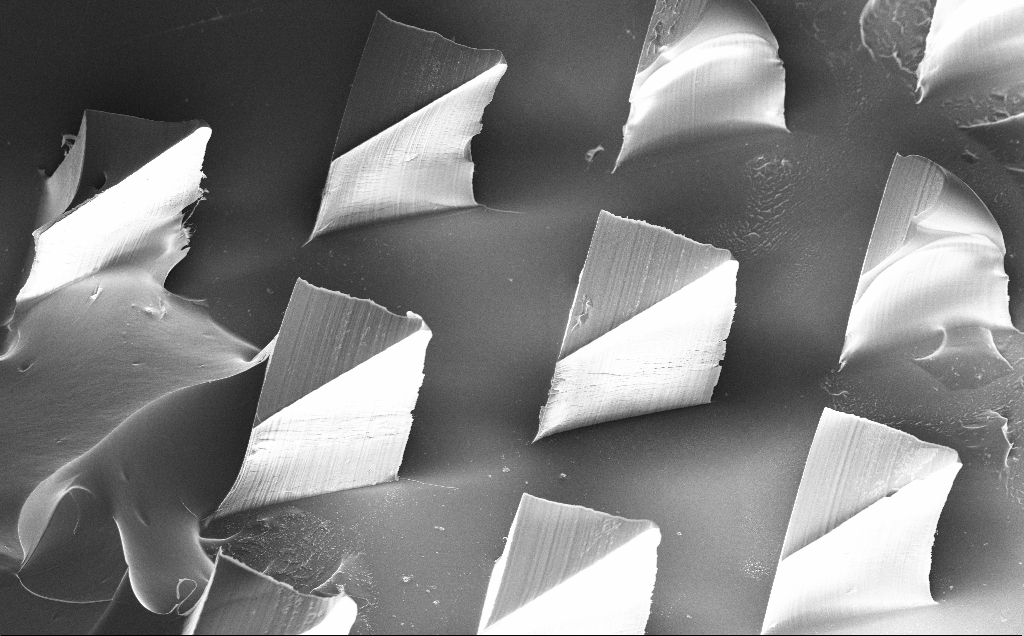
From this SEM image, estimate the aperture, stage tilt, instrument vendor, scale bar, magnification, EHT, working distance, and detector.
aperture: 30 µm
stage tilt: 20°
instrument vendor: Zeiss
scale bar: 100000 nm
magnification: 0.255 K X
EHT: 10 kV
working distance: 8 mm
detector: InLens